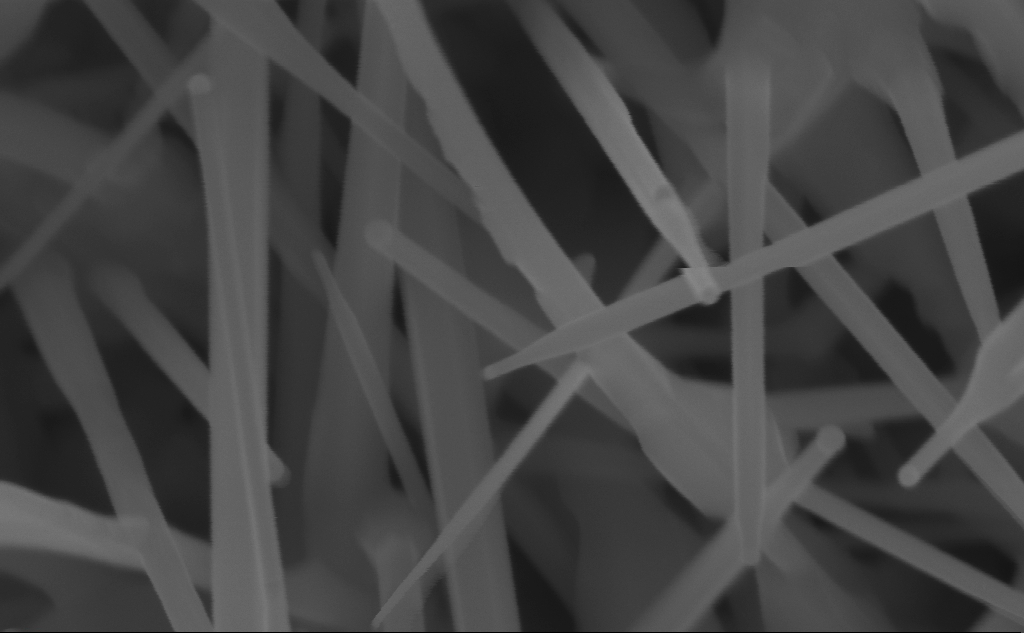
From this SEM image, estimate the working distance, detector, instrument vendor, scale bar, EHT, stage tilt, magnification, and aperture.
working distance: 7 mm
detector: InLens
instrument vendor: Zeiss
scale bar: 100 nm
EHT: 10 kV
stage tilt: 0°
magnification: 253.54 K X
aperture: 30 µm